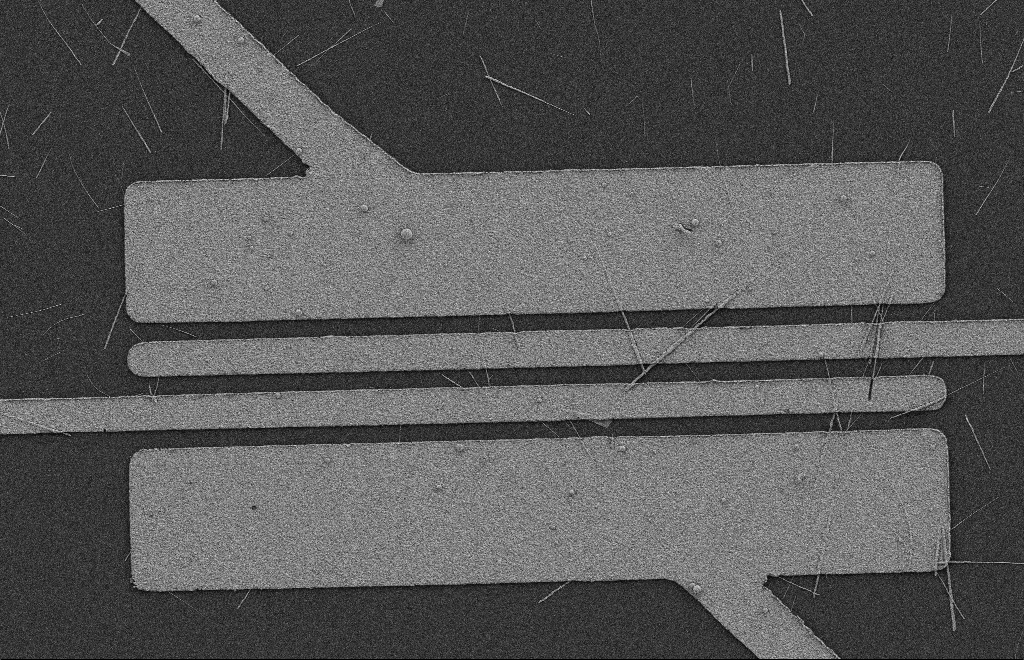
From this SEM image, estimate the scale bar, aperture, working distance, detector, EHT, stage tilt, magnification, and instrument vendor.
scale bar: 2000 nm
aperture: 20 µm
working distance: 9 mm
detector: SE2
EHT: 2 kV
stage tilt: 0°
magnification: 4.92 K X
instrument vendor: Zeiss